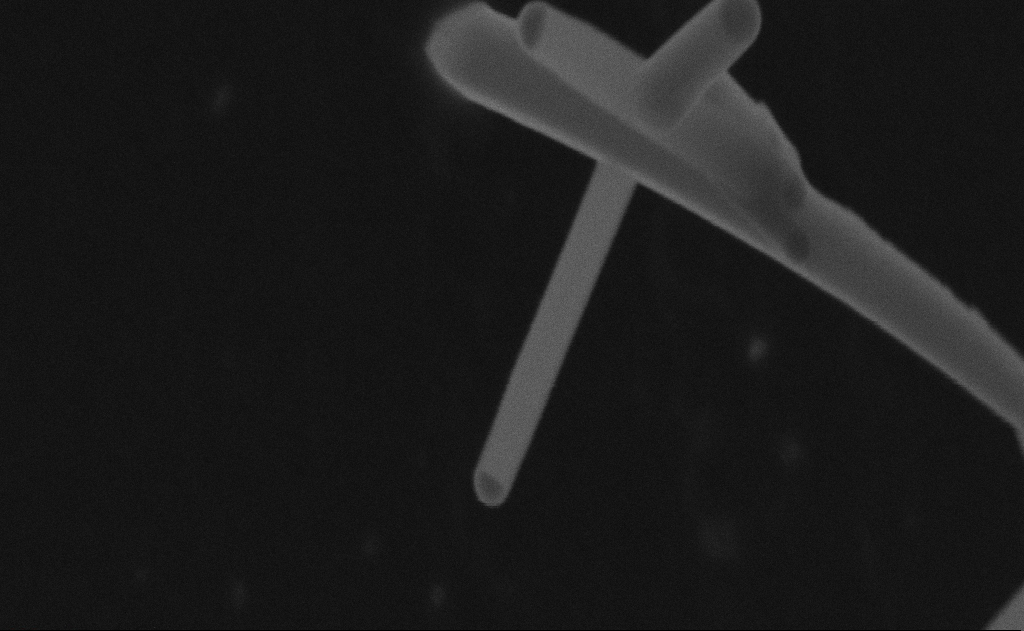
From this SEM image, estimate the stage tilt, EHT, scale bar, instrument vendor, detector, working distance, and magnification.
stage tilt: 0°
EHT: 25 kV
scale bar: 200 nm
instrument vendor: Zeiss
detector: SE2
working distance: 9 mm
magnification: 244 K X